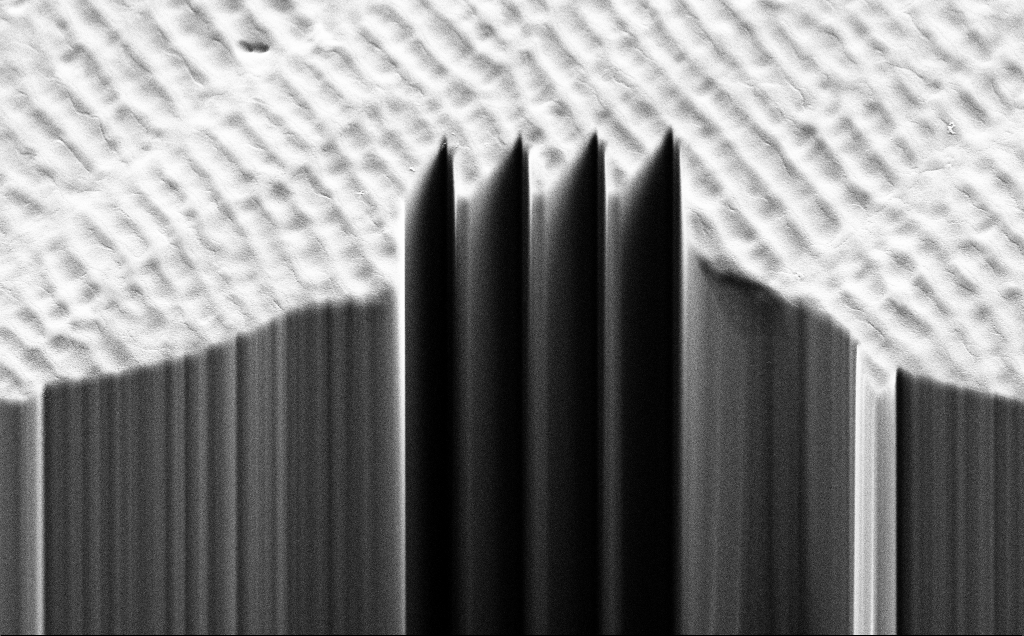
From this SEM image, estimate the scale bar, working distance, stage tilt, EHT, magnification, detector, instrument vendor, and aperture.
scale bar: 20000 nm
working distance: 13 mm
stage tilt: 45°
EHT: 10 kV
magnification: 2.4 K X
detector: SE2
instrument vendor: Zeiss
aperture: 30 µm